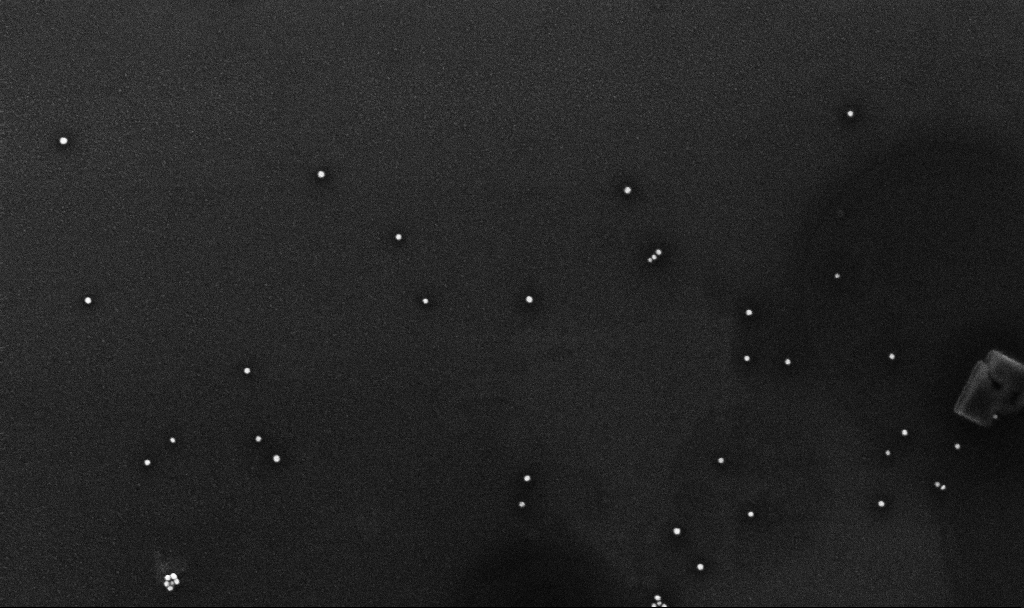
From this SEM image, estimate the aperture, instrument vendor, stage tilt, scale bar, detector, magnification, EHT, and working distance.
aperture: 30 µm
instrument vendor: Zeiss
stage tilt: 0°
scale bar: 200 nm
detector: InLens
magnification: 92.09 K X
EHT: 10 kV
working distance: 3.2 mm